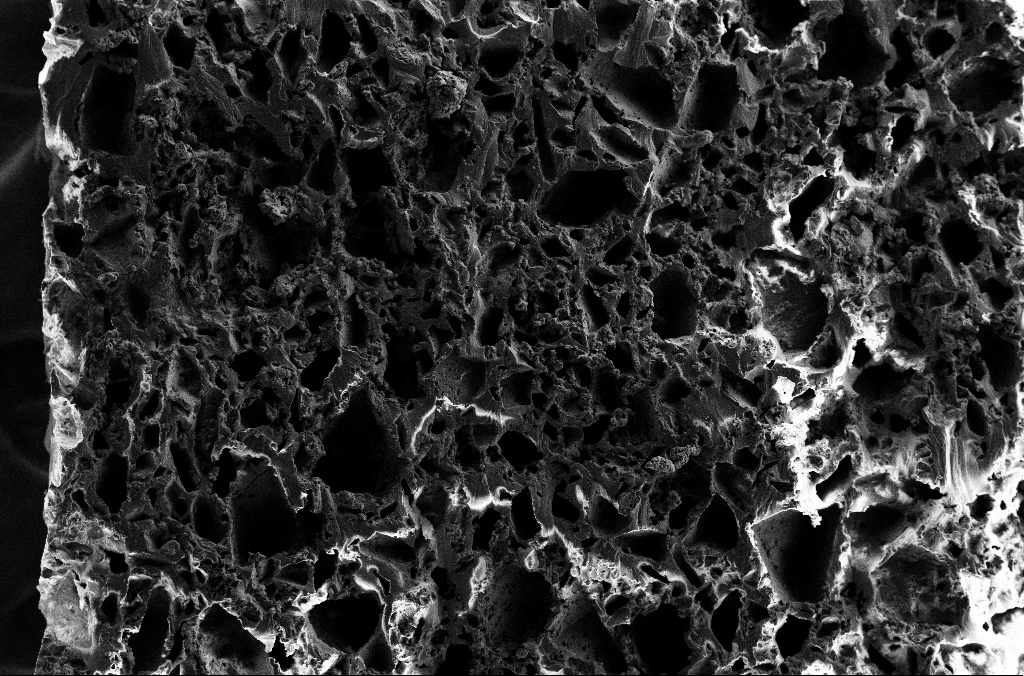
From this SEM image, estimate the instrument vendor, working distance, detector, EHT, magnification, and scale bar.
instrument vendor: Zeiss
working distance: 2.9 mm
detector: SE2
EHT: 5 kV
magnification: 0.2 K X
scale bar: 100000 nm